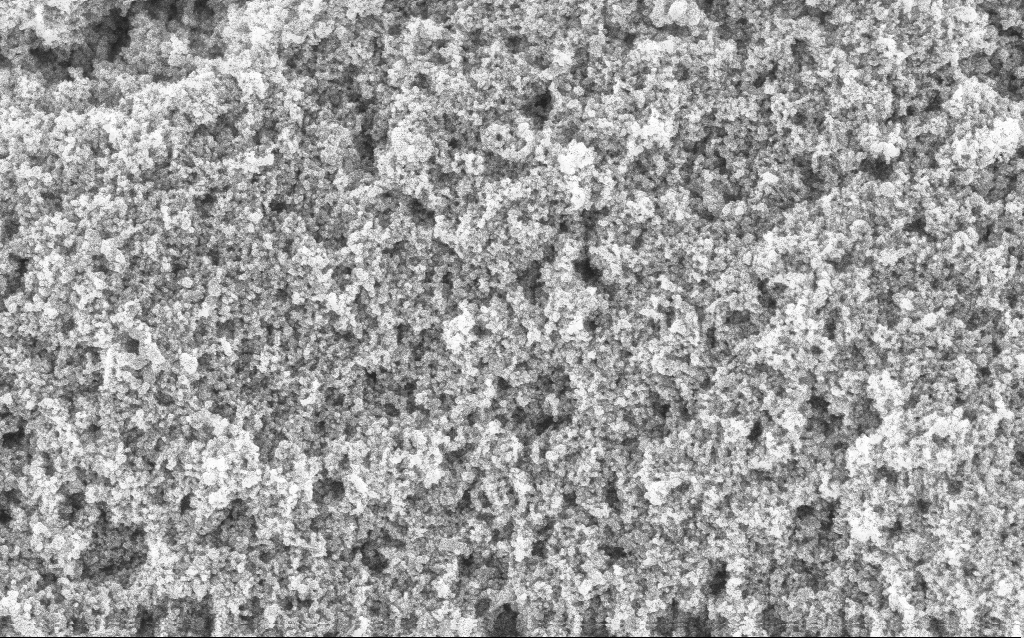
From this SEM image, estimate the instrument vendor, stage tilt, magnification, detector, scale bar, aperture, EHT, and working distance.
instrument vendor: Zeiss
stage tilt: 0°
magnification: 65.04 K X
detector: InLens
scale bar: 1000 nm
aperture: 30 µm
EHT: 5 kV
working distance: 4.4 mm